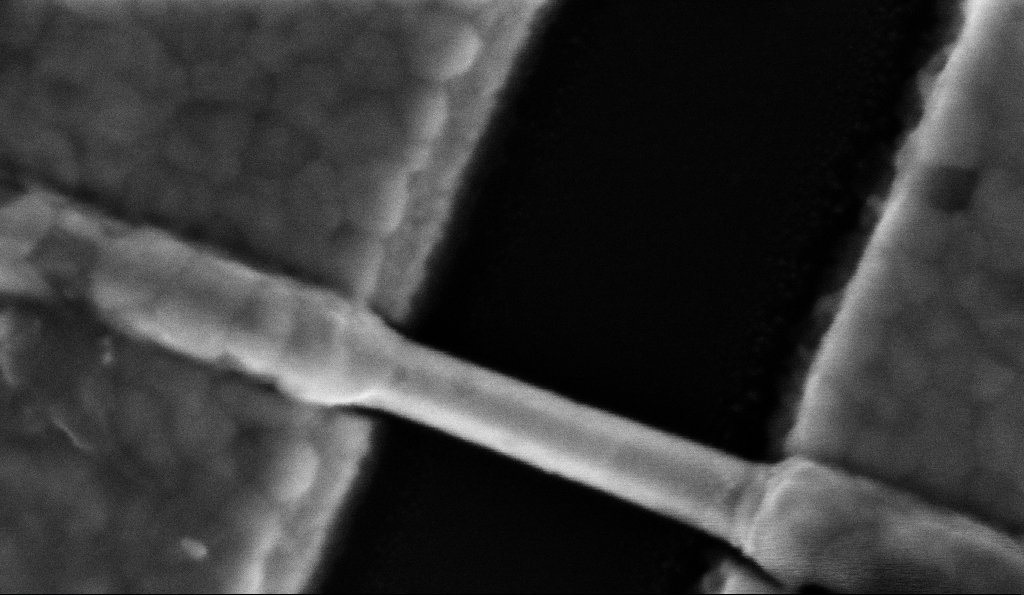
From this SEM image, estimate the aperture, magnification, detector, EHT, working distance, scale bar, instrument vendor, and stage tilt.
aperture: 30 µm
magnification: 300 K X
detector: InLens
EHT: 5 kV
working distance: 8.5 mm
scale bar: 200 nm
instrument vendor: Zeiss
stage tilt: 0°